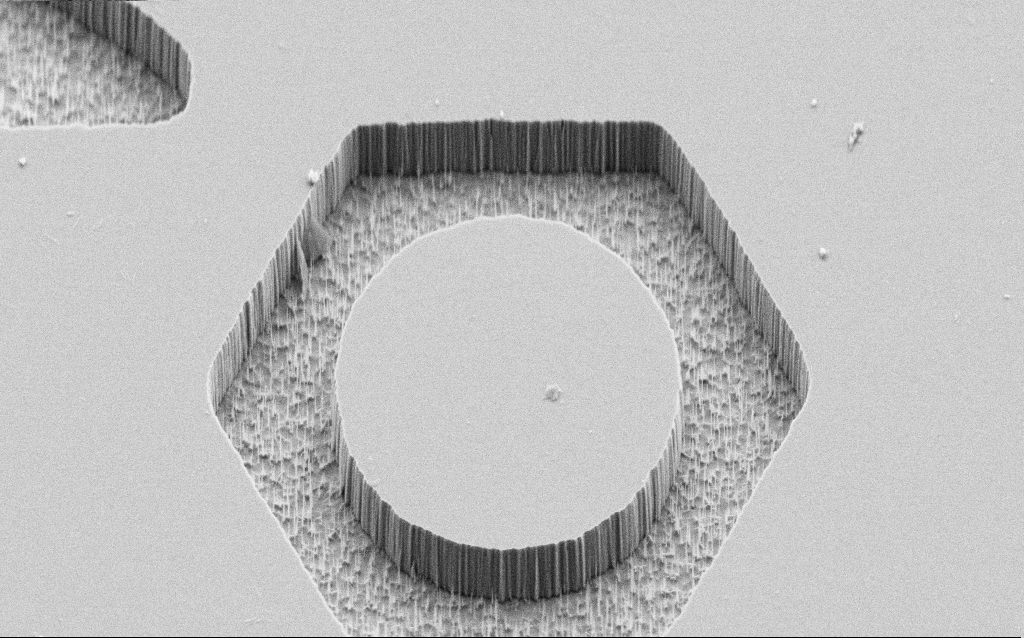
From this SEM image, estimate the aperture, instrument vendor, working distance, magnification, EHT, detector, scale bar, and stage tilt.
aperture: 30 µm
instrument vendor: Zeiss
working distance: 7 mm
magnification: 4 K X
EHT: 5 kV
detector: SE2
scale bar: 10000 nm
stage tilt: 45°